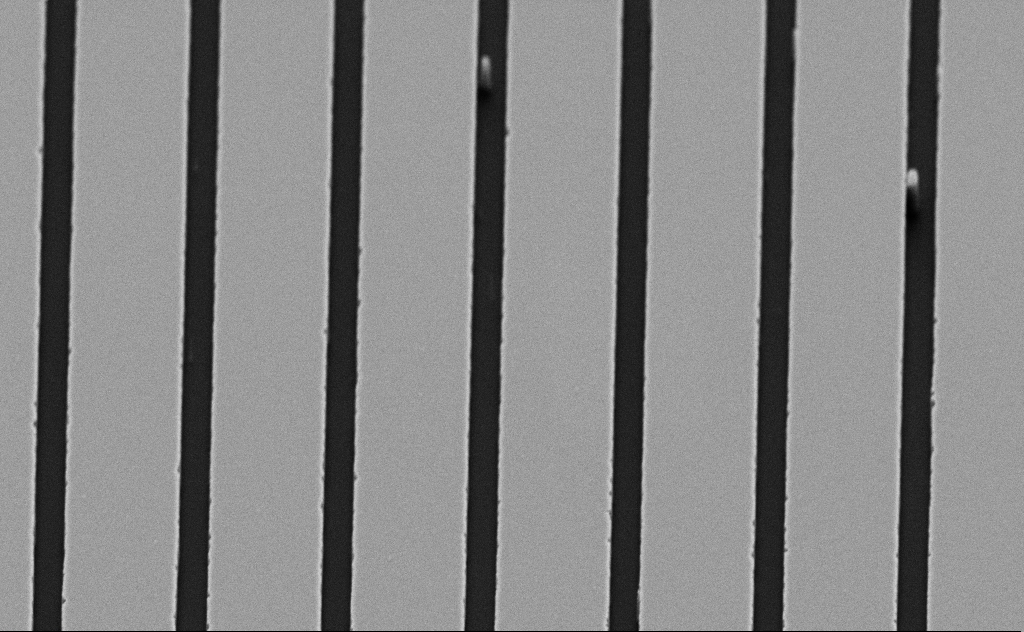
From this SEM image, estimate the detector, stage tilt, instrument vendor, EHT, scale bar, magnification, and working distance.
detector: SE2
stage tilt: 45°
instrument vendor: Zeiss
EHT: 5 kV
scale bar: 2000 nm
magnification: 13.27 K X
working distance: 9 mm